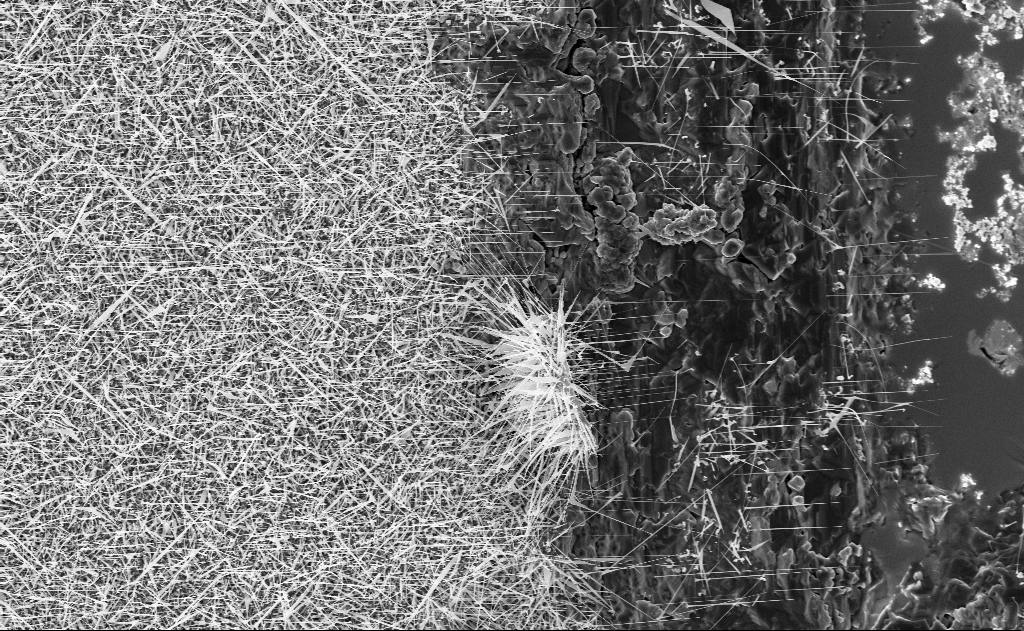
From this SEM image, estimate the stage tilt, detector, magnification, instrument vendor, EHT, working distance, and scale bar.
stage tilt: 0°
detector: InLens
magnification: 5 K X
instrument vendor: Zeiss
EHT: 10 kV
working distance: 11 mm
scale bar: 10000 nm